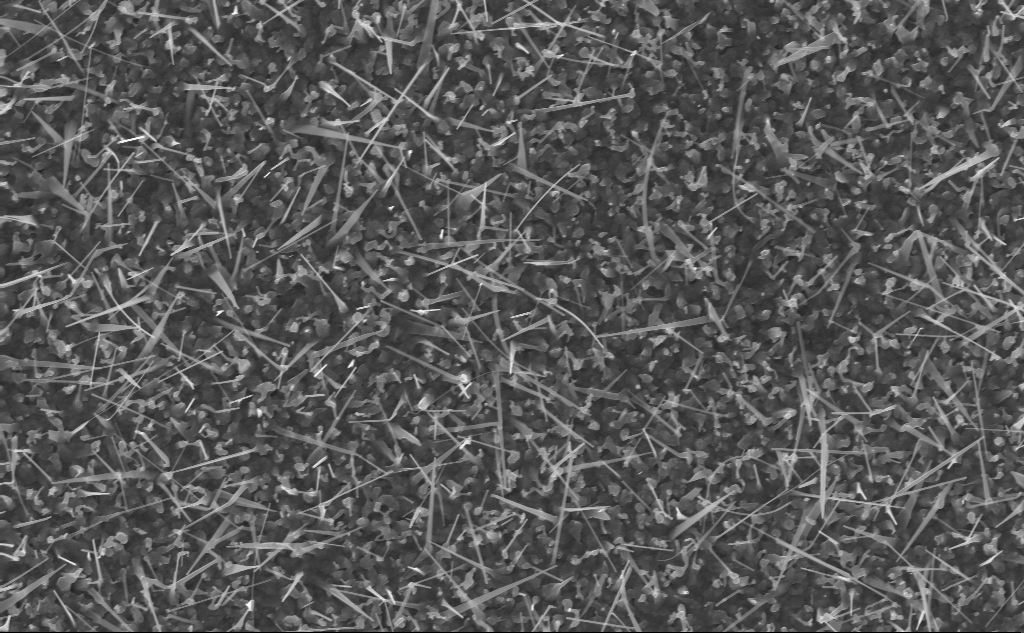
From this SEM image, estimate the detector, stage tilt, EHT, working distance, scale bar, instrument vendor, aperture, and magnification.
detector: InLens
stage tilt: -0°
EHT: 10 kV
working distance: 5 mm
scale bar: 1000 nm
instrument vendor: Zeiss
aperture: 30 µm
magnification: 20 K X